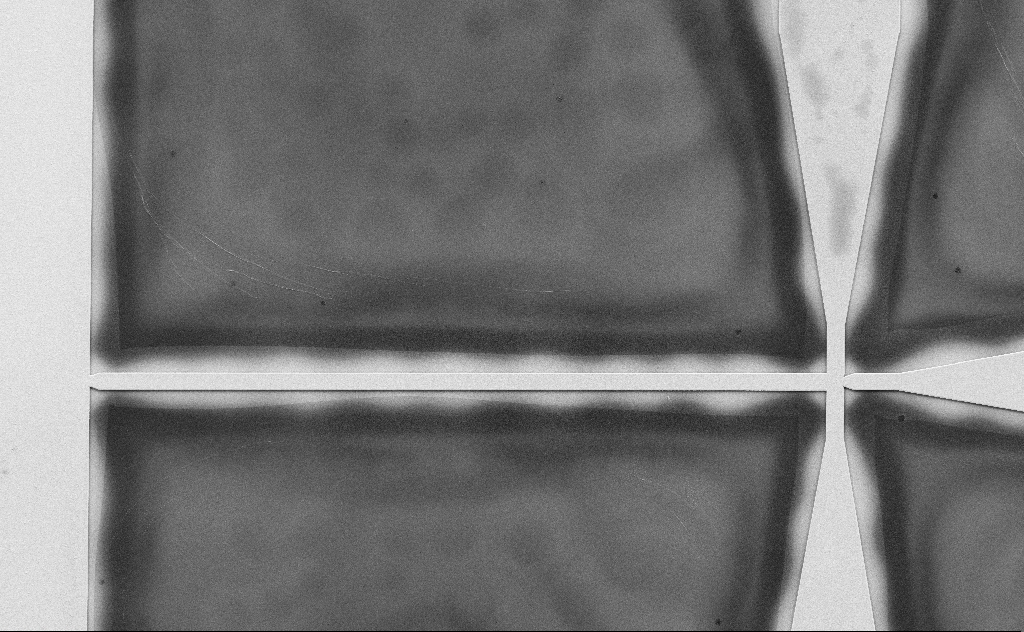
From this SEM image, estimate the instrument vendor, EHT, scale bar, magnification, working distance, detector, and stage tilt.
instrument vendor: Zeiss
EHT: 10 kV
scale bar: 100000 nm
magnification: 0.541 K X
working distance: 11 mm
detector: SE2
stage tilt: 0°